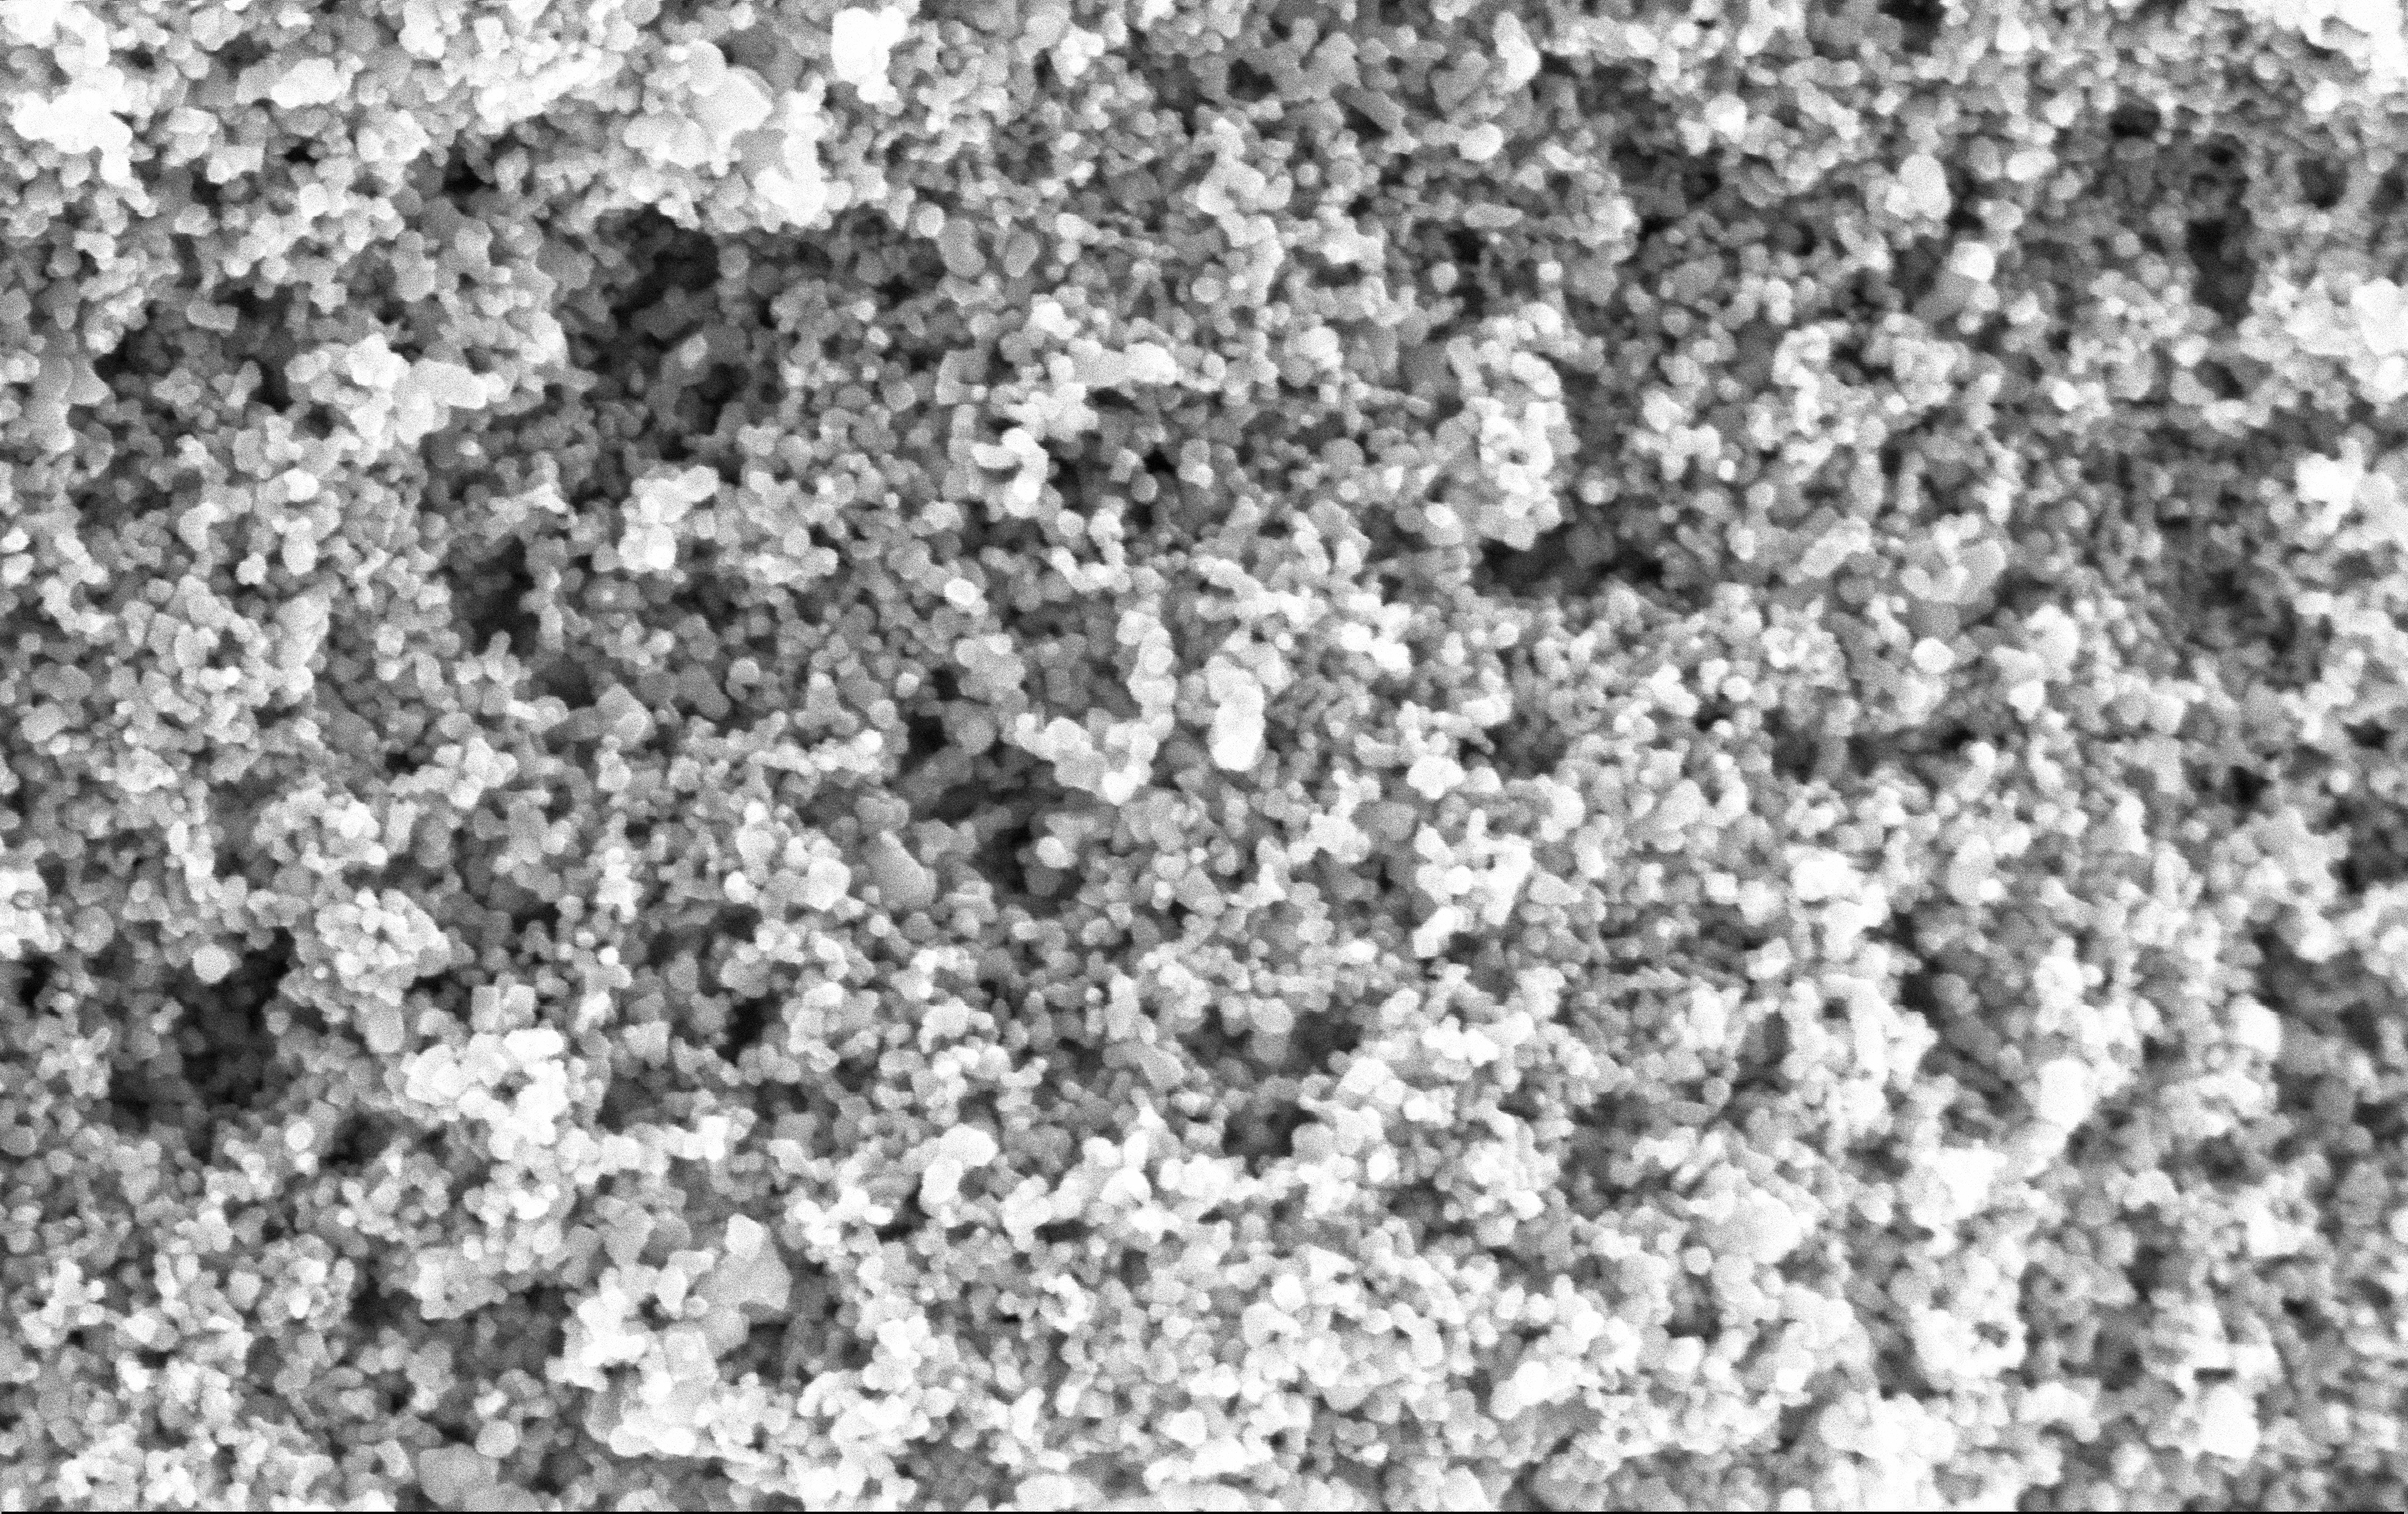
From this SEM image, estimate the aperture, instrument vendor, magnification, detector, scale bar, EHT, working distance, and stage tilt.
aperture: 30 µm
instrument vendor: Zeiss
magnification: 135 K X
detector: InLens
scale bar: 100 nm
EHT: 5 kV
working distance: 4.9 mm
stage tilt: -0°